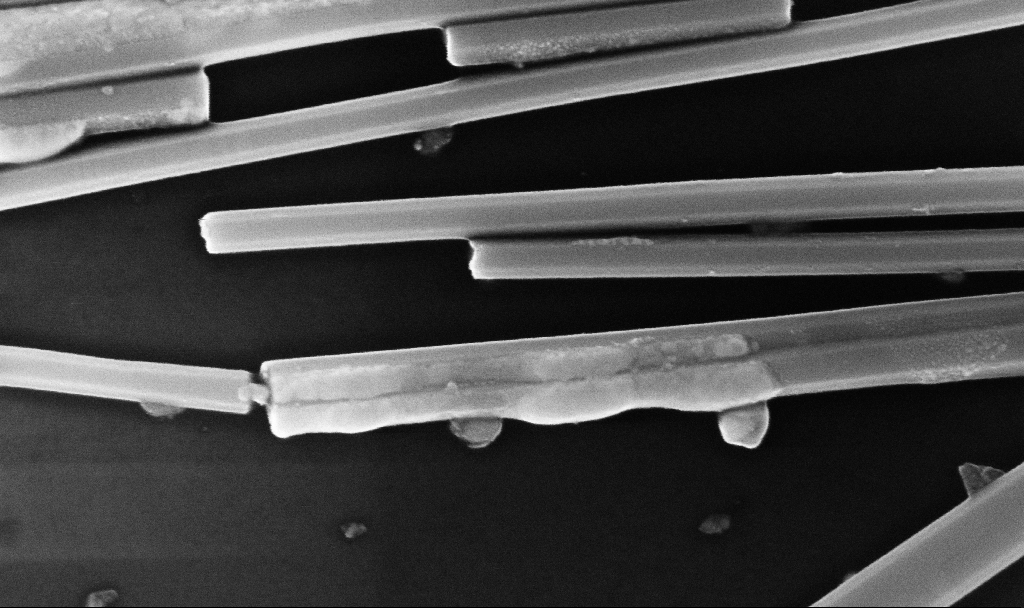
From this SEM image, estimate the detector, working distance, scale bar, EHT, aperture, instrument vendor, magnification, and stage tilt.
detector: InLens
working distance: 6.7 mm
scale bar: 200 nm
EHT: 10 kV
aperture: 30 µm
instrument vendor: Zeiss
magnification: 150 K X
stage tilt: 0°